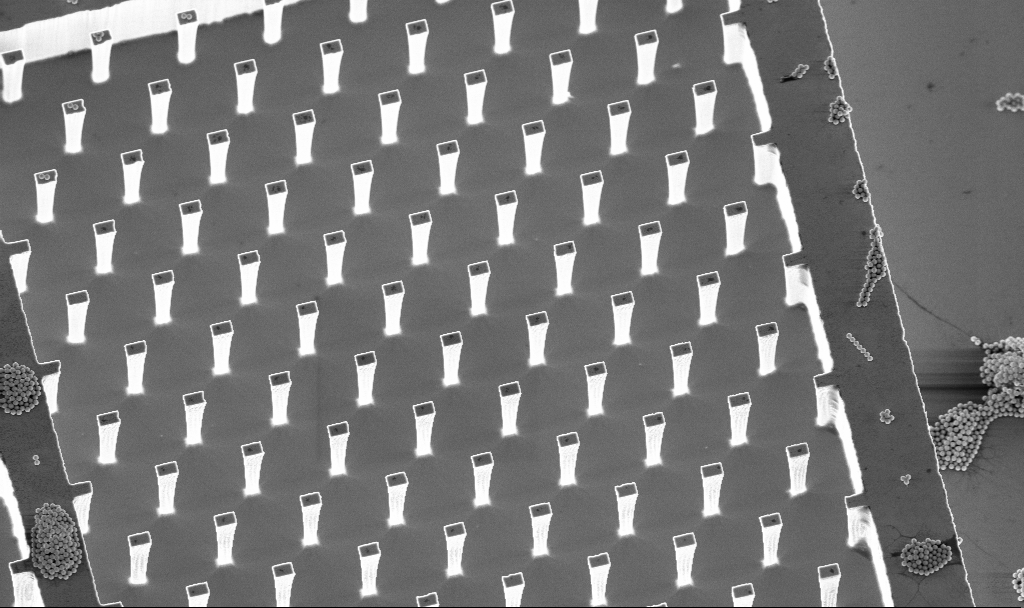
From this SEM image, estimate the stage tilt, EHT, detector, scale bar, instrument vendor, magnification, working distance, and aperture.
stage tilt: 20°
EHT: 5 kV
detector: InLens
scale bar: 20000 nm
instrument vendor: Zeiss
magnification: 2.71 K X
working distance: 4.7 mm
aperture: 30 µm